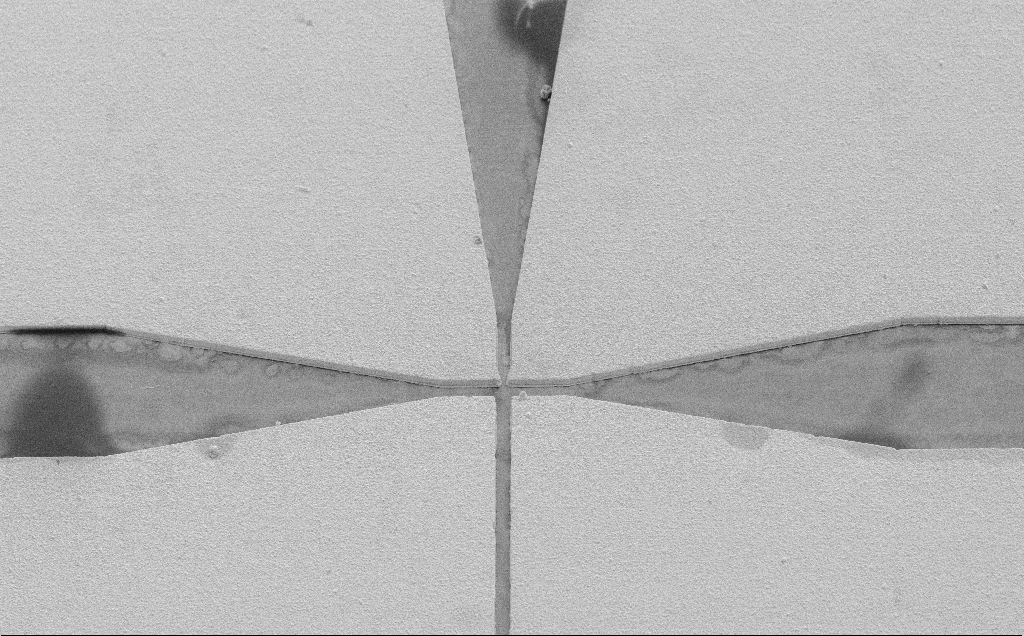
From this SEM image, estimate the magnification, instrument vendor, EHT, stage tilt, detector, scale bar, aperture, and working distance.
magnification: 0.609 K X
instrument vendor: Zeiss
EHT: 10 kV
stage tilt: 45°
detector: SE2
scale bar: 100000 nm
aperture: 30 µm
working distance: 11 mm